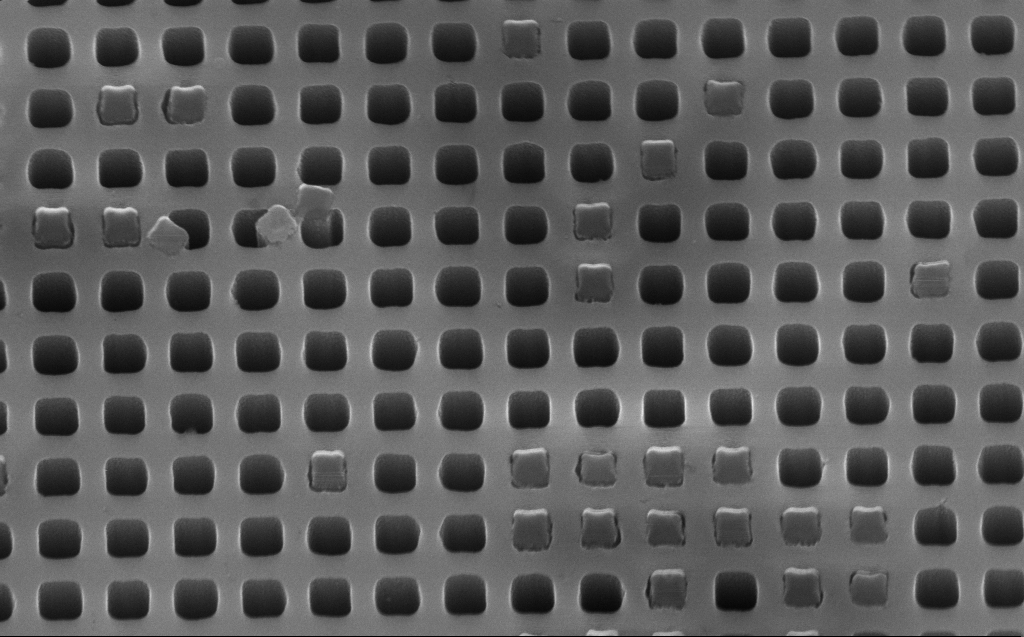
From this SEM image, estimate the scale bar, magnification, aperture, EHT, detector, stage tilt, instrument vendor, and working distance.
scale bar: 1000 nm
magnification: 50.04 K X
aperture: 30 µm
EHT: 10 kV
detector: InLens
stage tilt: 45°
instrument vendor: Zeiss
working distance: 7 mm